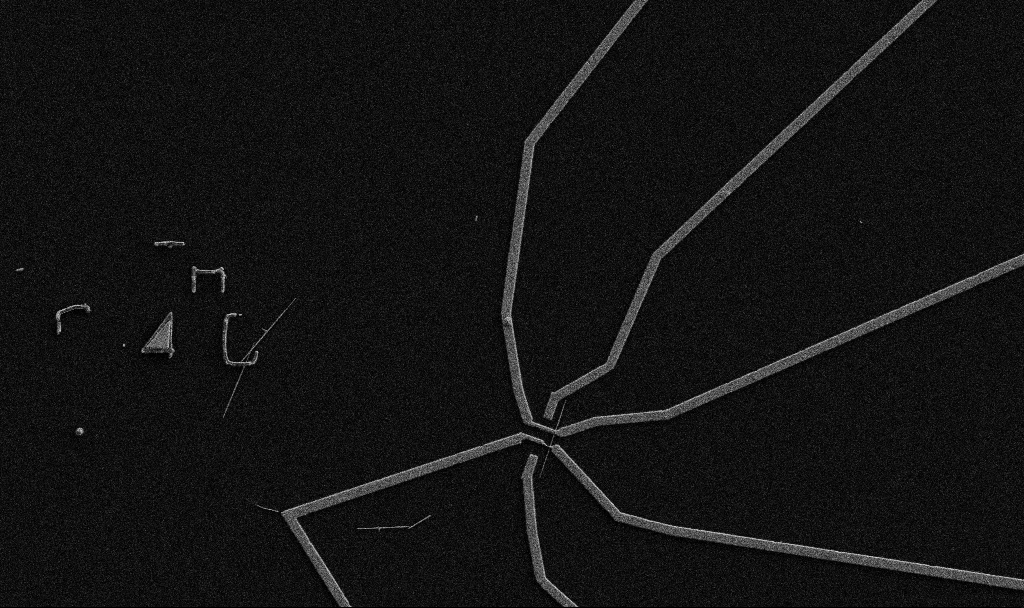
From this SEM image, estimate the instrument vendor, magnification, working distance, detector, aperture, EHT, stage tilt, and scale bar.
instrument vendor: Zeiss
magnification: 5 K X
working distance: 10.7 mm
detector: SE2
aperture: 30 µm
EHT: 5 kV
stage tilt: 0°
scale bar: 10000 nm